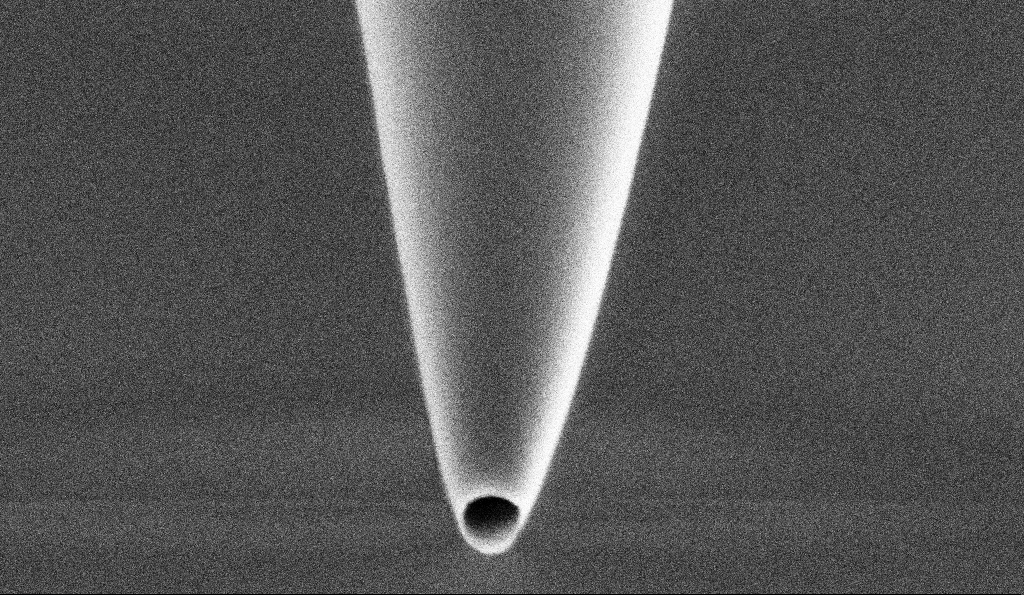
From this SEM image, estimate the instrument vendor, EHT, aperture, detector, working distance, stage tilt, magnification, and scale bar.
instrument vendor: Zeiss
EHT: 1 kV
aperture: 30 µm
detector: SE2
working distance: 6.4 mm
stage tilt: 45°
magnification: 100 K X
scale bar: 200 nm